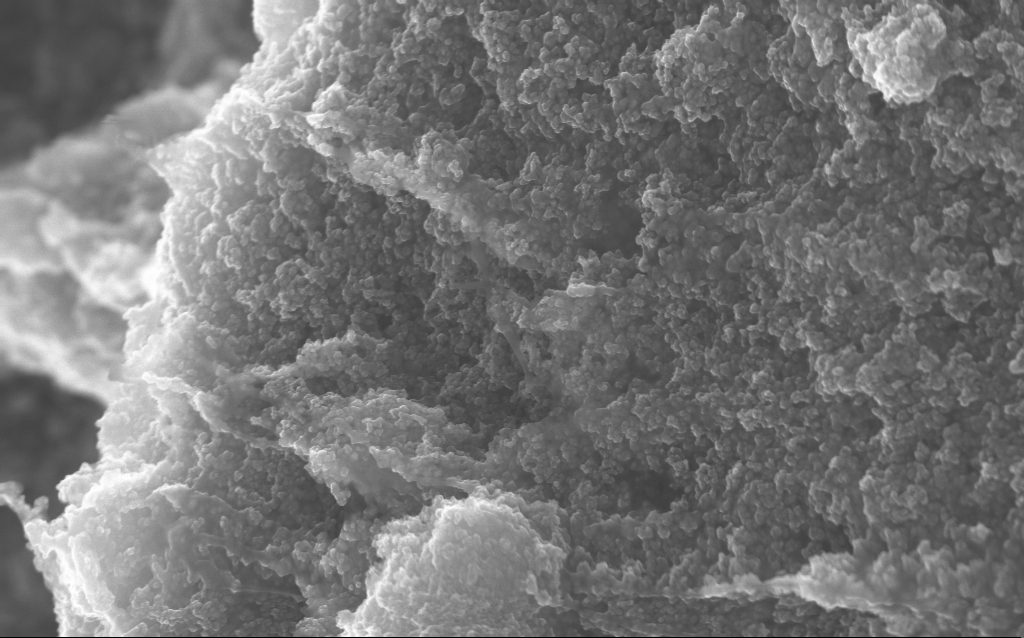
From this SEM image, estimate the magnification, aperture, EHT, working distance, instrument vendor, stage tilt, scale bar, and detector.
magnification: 65.04 K X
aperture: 30 µm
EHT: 10 kV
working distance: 2.7 mm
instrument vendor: Zeiss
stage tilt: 0°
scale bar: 1000 nm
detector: InLens